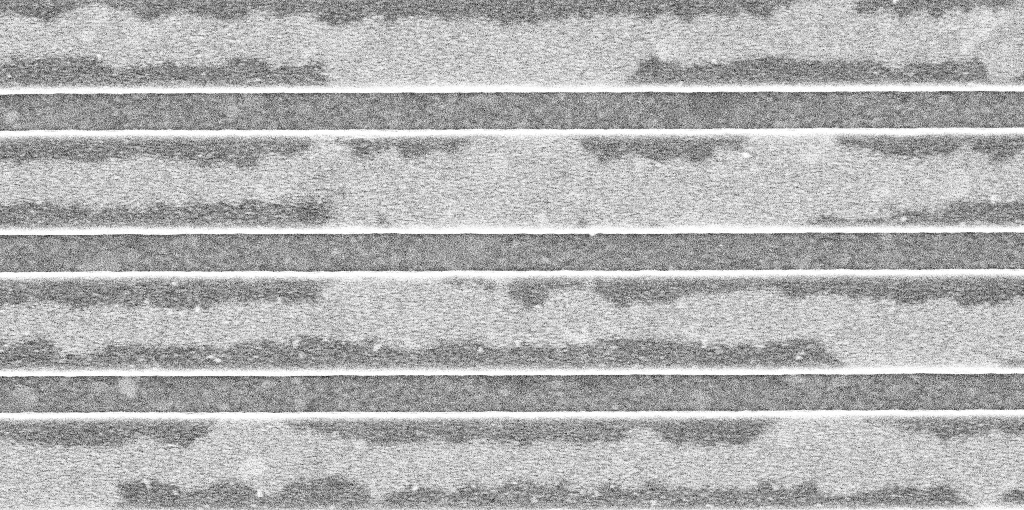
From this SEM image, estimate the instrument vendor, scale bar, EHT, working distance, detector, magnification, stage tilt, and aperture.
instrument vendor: Zeiss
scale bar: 1000 nm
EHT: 5 kV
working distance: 3.1 mm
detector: InLens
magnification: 58.05 K X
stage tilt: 0°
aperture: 30 µm